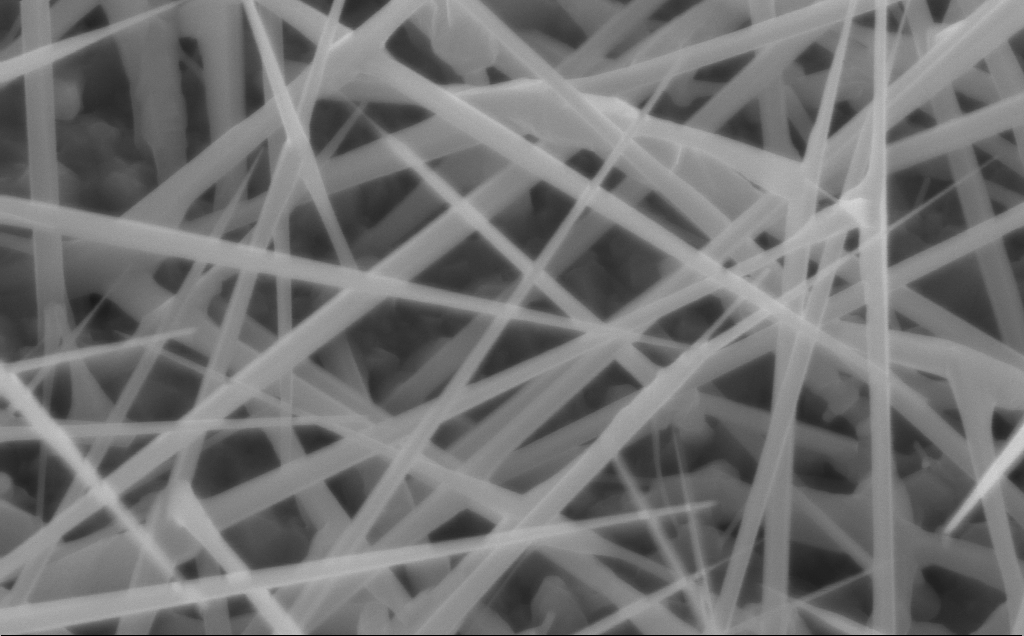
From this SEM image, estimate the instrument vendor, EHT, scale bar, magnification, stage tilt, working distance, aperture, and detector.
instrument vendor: Zeiss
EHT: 10 kV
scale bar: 200 nm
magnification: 80 K X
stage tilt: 30°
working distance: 5 mm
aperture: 30 µm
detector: InLens